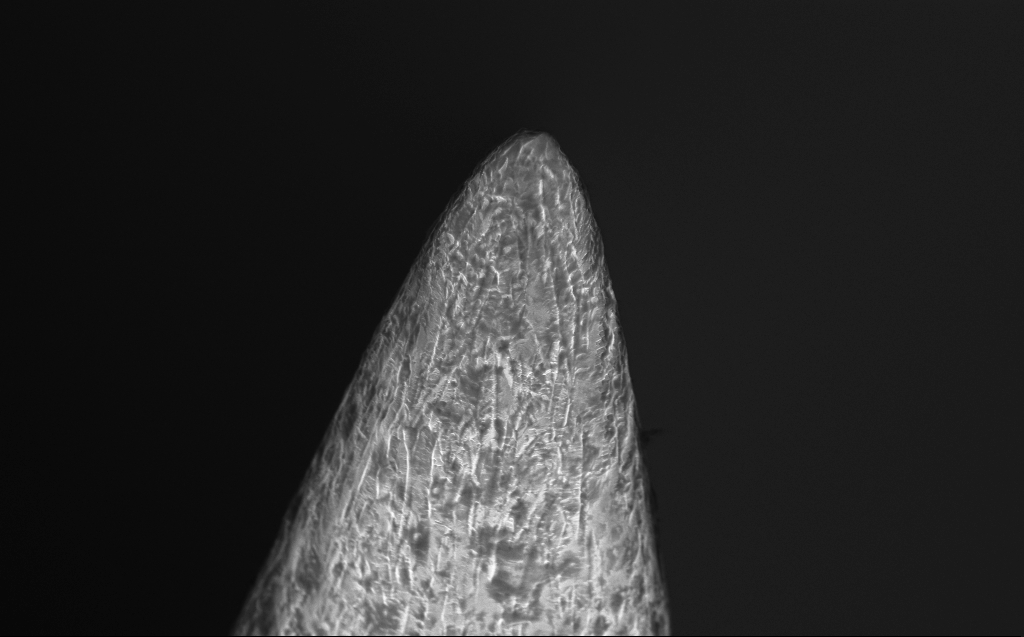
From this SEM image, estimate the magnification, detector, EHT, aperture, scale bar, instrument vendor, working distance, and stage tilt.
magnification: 15.28 K X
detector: InLens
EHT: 10 kV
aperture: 30 µm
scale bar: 2000 nm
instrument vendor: Zeiss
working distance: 5 mm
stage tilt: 40°